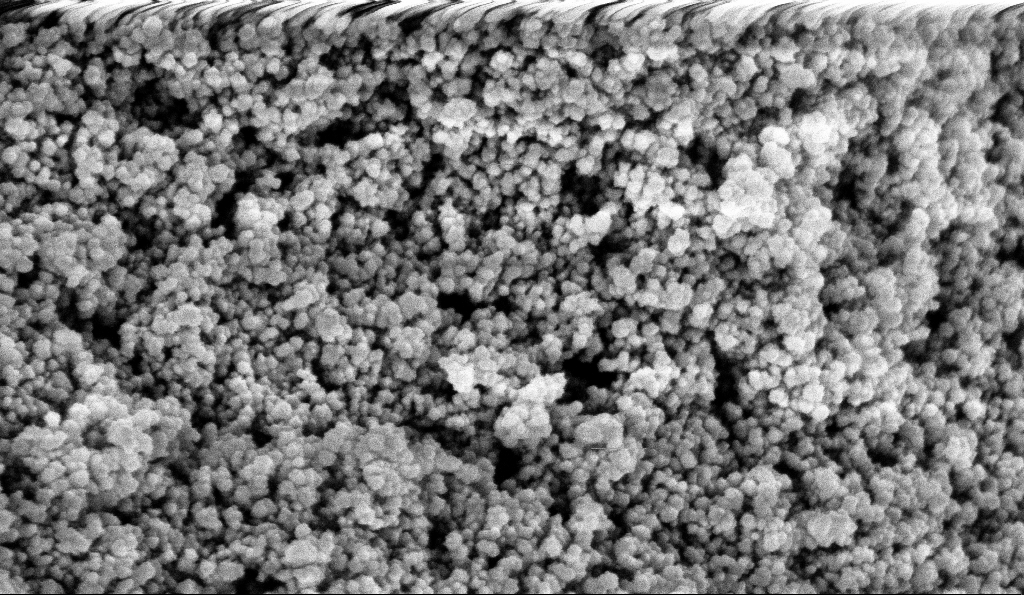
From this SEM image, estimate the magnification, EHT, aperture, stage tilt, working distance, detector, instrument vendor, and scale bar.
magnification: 135 K X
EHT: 5 kV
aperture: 30 µm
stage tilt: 0°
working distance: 5.9 mm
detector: InLens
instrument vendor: Zeiss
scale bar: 100 nm